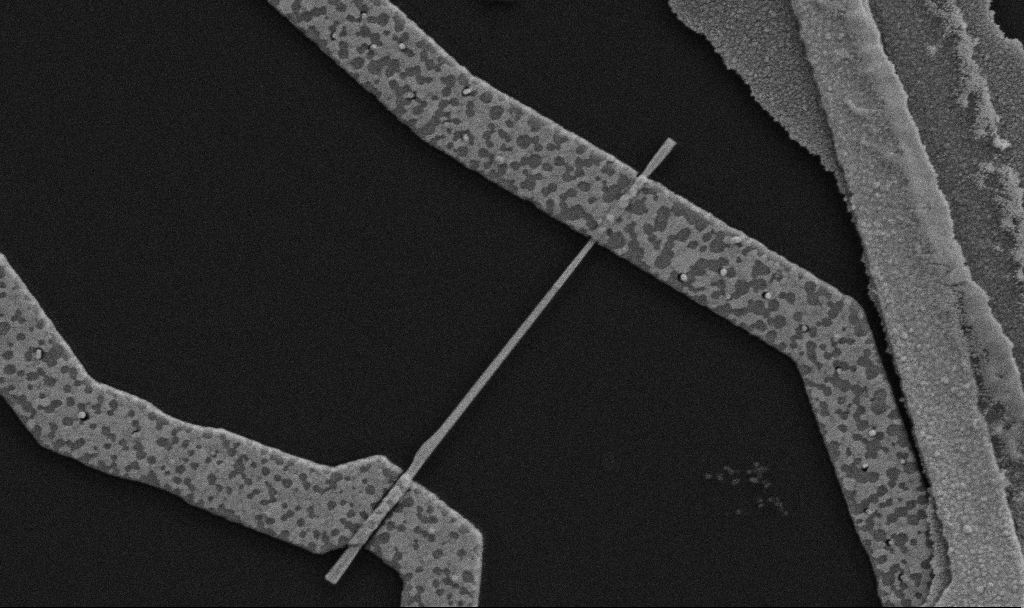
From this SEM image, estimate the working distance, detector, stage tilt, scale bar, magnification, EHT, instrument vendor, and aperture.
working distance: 10.7 mm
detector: SE2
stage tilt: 0°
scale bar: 1000 nm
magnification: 30 K X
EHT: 5 kV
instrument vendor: Zeiss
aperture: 30 µm